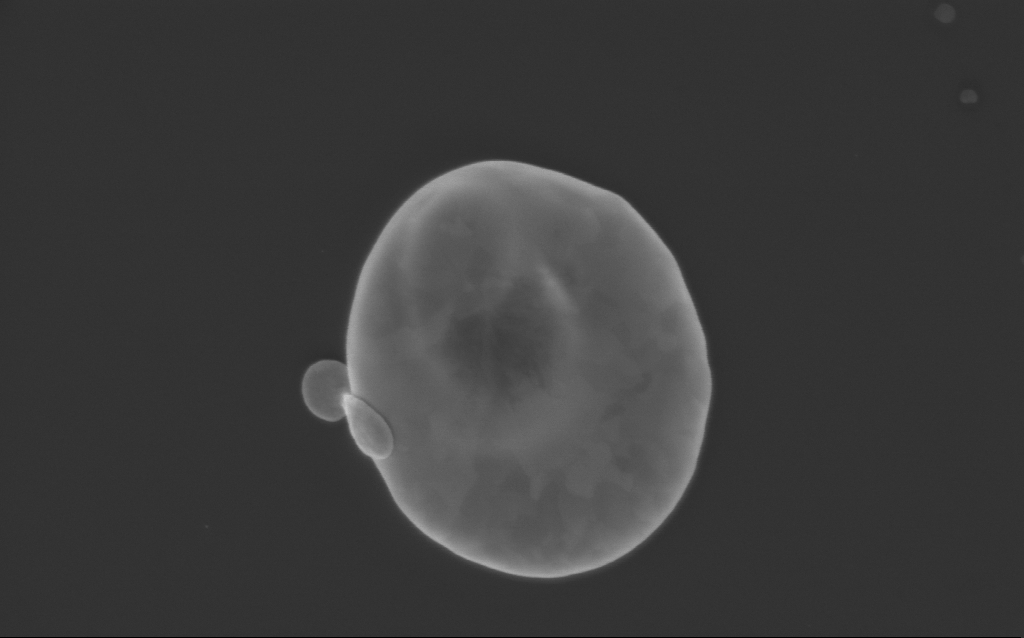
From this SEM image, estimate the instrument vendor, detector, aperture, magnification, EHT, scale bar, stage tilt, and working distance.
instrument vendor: Zeiss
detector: InLens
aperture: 30 µm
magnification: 78.44 K X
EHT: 3 kV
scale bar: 200 nm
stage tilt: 0°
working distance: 4 mm